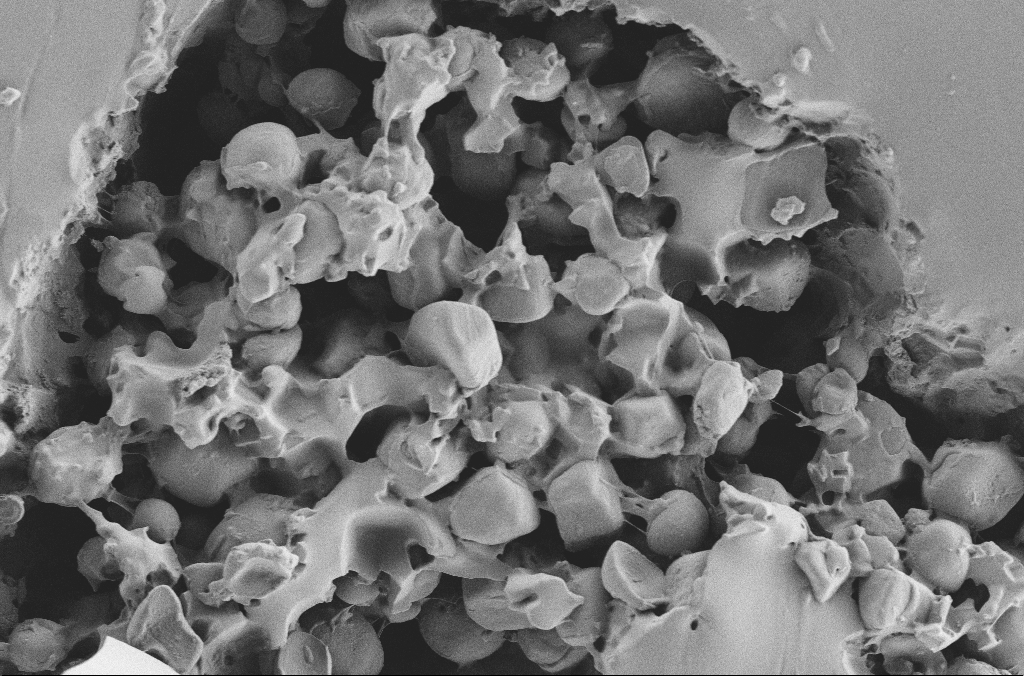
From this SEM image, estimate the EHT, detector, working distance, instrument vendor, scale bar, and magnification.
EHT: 2 kV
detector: SE2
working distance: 3.6 mm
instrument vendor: Zeiss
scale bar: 20000 nm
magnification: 2.5 K X